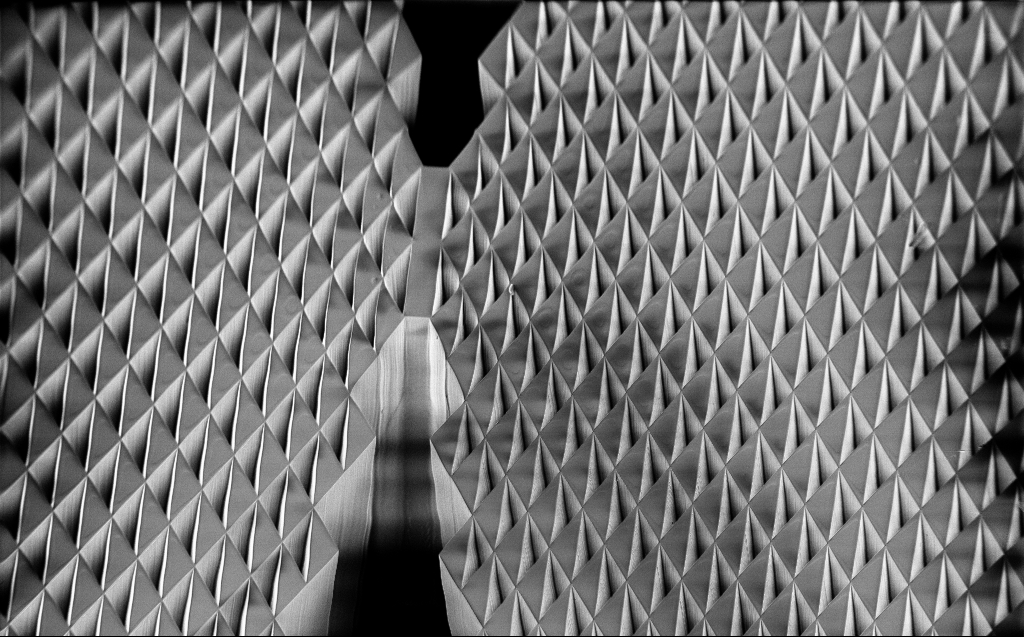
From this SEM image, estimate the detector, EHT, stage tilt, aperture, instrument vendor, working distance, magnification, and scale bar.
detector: InLens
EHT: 1 kV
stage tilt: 45°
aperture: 30 µm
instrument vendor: Zeiss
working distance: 5 mm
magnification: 0.291 K X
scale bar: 100000 nm